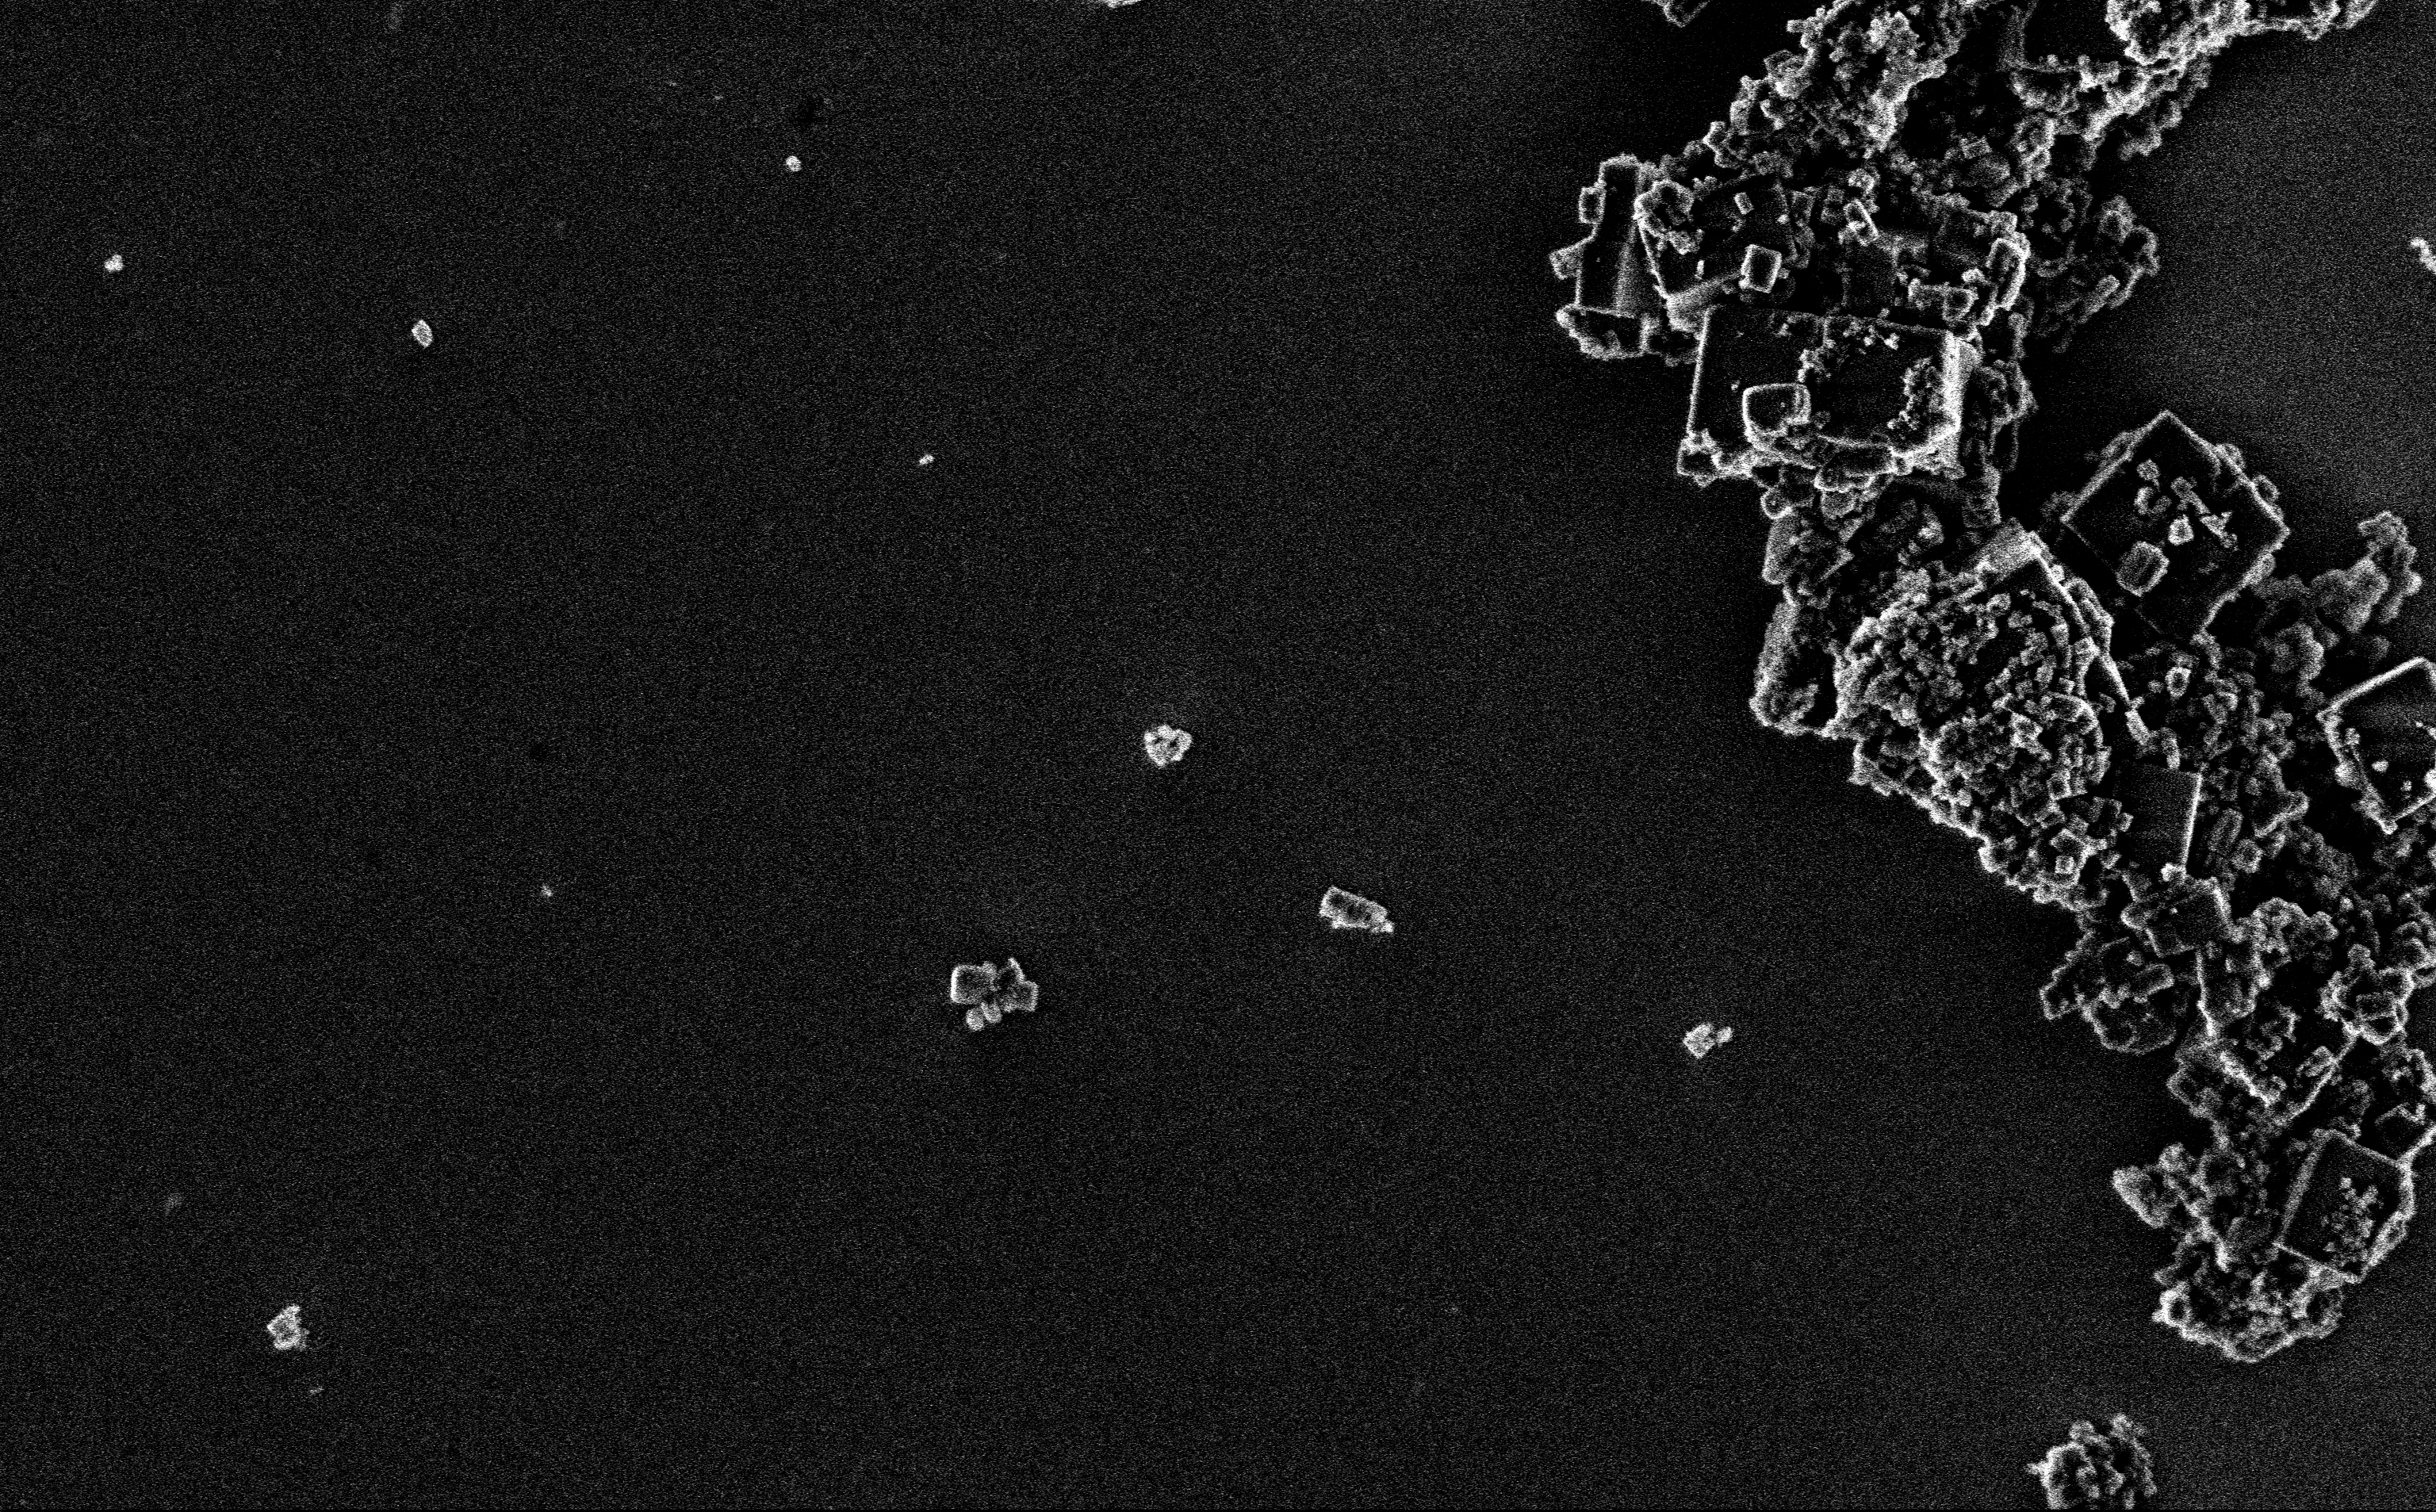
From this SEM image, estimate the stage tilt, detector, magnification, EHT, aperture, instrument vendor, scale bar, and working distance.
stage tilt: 0°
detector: InLens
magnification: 12.85 K X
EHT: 3 kV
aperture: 30 µm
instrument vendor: Zeiss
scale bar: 2000 nm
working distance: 3 mm